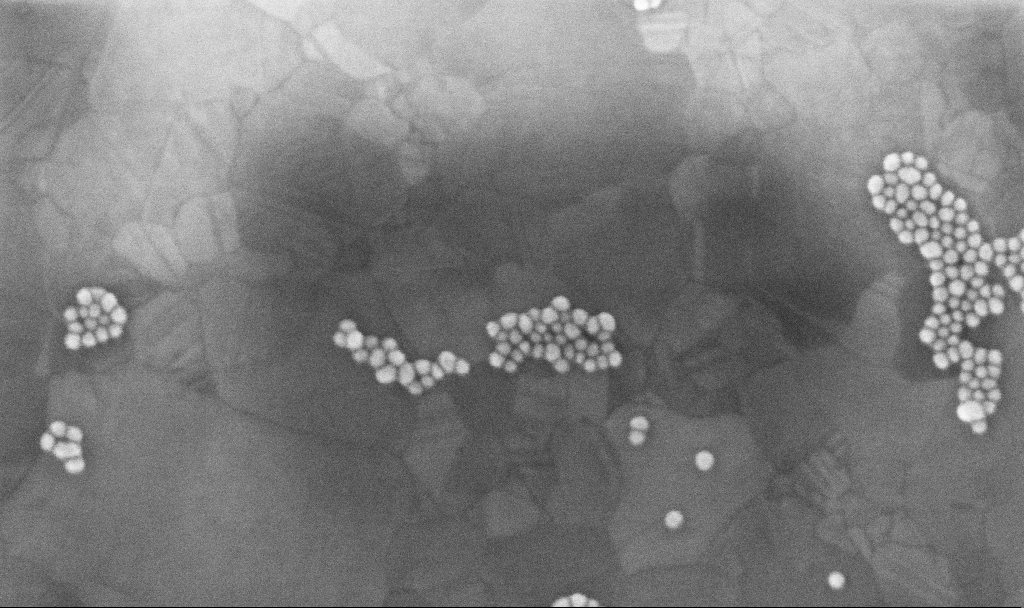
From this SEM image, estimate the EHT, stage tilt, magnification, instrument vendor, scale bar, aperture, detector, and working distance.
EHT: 10 kV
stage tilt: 0°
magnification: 300 K X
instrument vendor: Zeiss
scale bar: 100 nm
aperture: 30 µm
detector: InLens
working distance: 3.4 mm